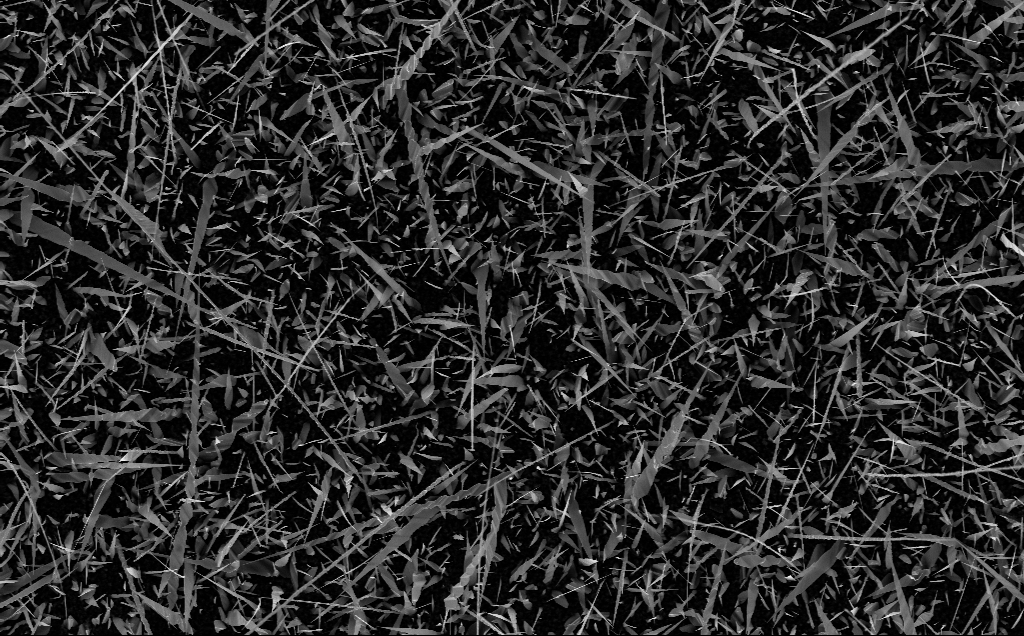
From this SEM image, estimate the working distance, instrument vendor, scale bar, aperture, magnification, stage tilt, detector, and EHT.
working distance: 6 mm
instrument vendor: Zeiss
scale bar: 10000 nm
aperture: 30 µm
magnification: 5 K X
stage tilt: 0°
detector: InLens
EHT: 10 kV